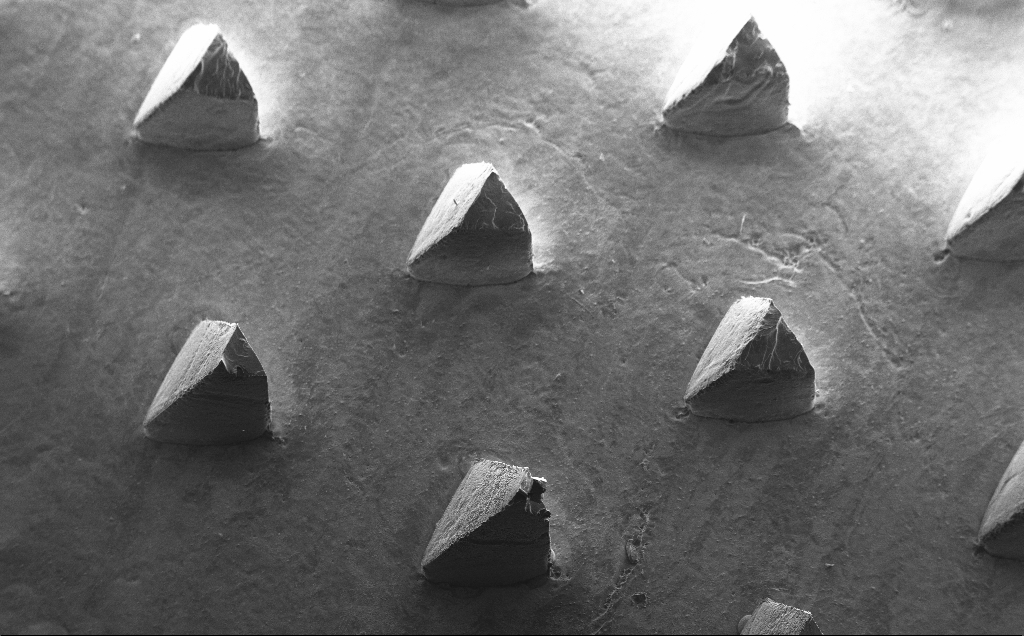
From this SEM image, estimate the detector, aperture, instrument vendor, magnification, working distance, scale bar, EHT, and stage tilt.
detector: SE2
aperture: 30 µm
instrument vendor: Zeiss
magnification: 0.082 K X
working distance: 9 mm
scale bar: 200000 nm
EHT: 10 kV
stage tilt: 30°